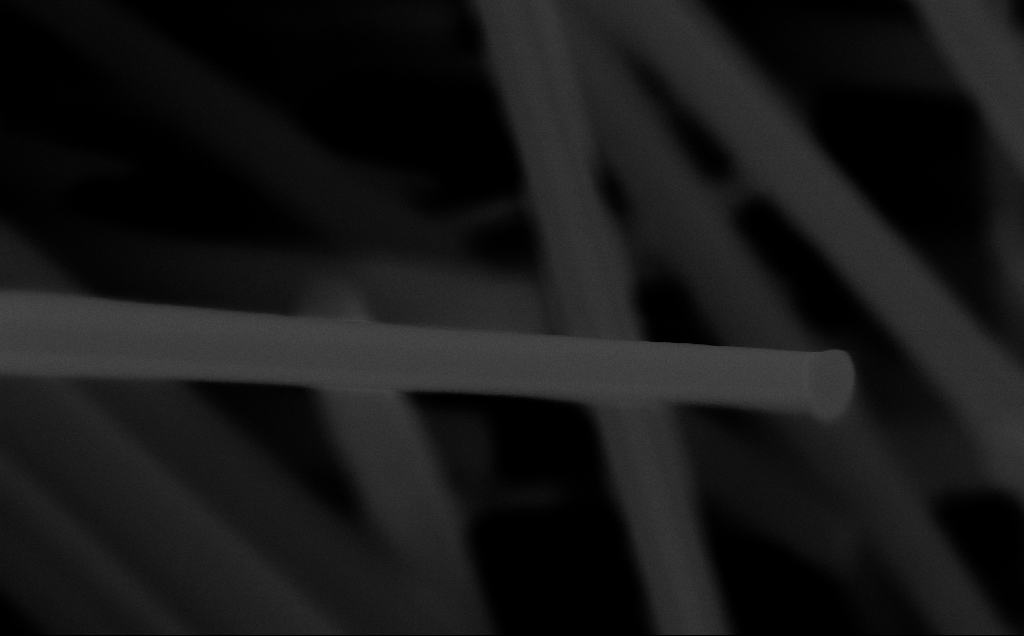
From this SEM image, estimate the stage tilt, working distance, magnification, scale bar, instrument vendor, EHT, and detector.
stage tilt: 0°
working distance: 6 mm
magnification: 188.51 K X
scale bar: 200 nm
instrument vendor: Zeiss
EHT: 10 kV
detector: InLens